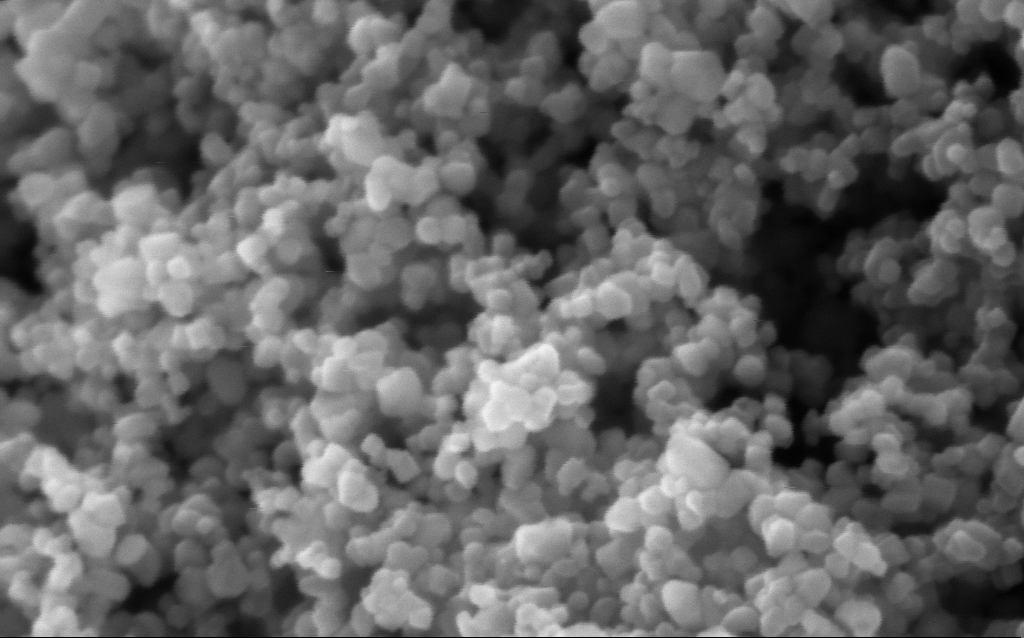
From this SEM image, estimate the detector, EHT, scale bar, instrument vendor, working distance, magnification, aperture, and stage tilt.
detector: InLens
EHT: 5 kV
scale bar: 100 nm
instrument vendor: Zeiss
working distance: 3.4 mm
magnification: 416 K X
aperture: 30 µm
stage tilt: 0°